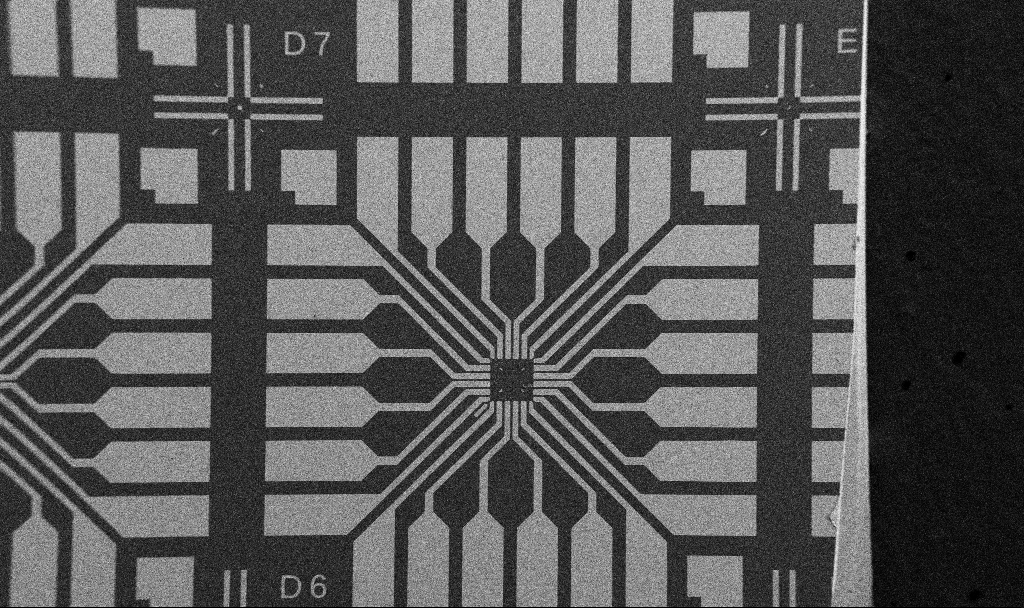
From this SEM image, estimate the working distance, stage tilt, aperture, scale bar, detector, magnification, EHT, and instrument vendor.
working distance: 10.7 mm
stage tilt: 0°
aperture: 30 µm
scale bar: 200000 nm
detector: SE2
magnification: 0.1 K X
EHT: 5 kV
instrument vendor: Zeiss